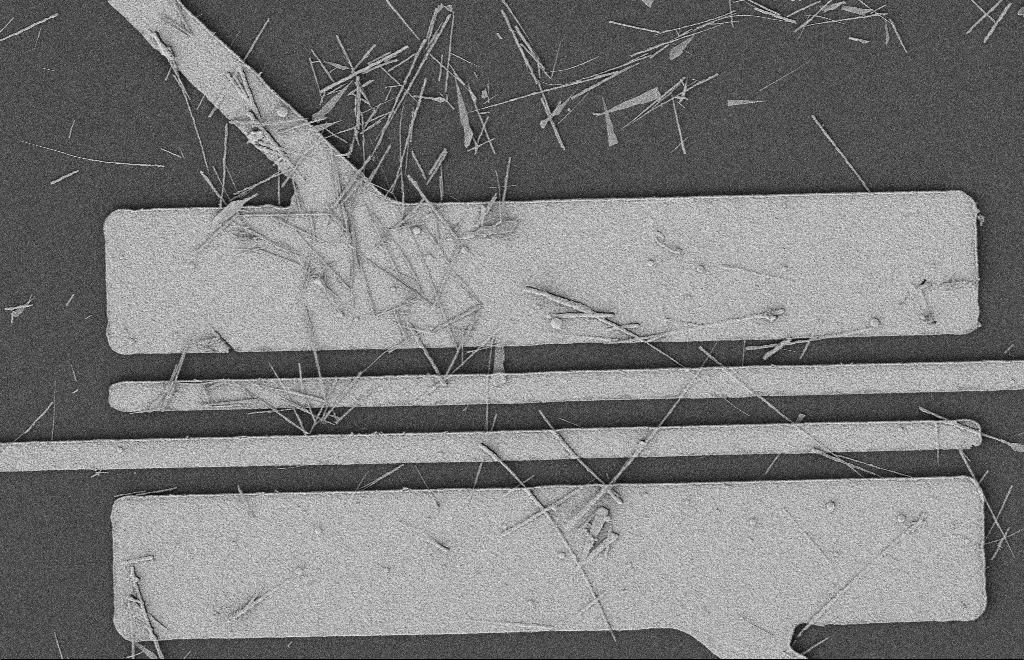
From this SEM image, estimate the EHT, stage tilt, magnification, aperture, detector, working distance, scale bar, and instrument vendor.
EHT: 2 kV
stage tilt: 0°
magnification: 5.22 K X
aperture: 20 µm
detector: SE2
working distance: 12 mm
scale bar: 2000 nm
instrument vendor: Zeiss